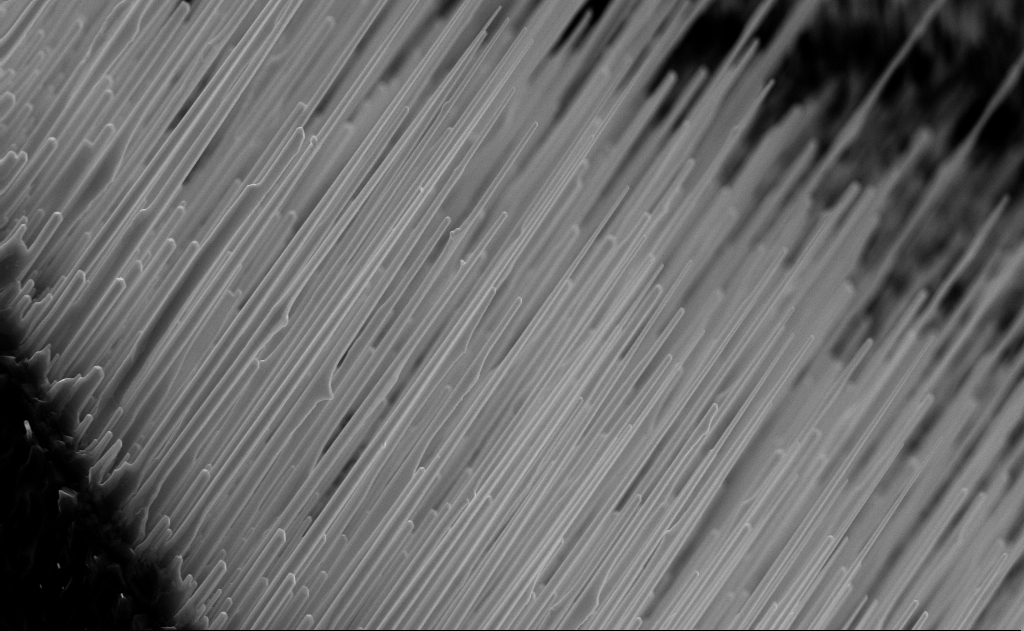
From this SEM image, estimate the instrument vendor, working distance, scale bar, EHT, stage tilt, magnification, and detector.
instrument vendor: Zeiss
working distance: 6 mm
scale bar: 2000 nm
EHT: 10 kV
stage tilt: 0°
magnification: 20 K X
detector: InLens